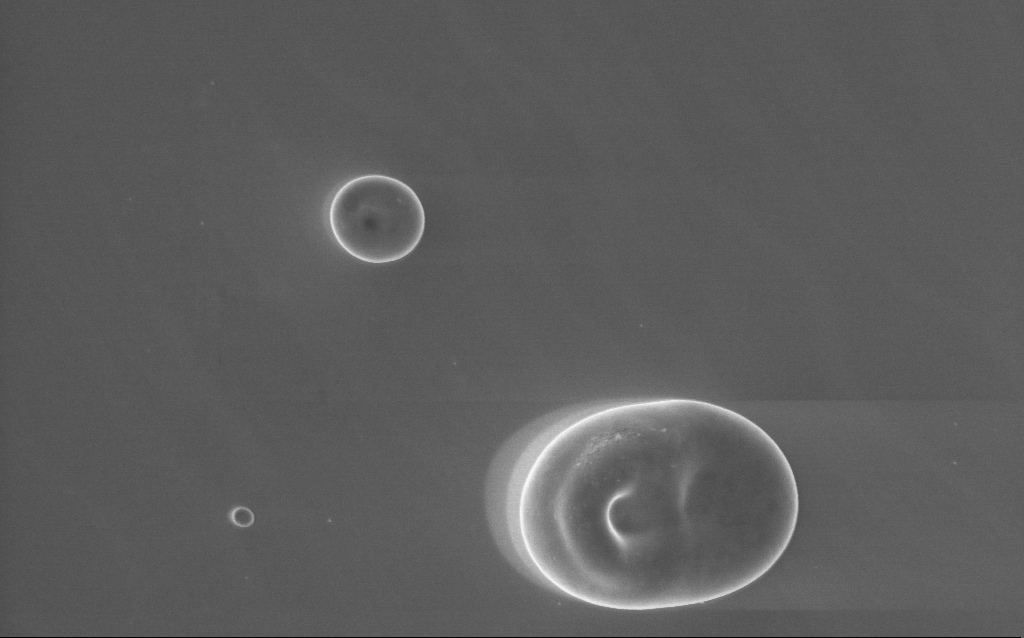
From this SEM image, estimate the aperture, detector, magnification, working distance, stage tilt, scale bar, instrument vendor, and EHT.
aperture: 30 µm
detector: InLens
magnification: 20 K X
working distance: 3 mm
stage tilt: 0°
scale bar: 2000 nm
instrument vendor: Zeiss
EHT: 5 kV